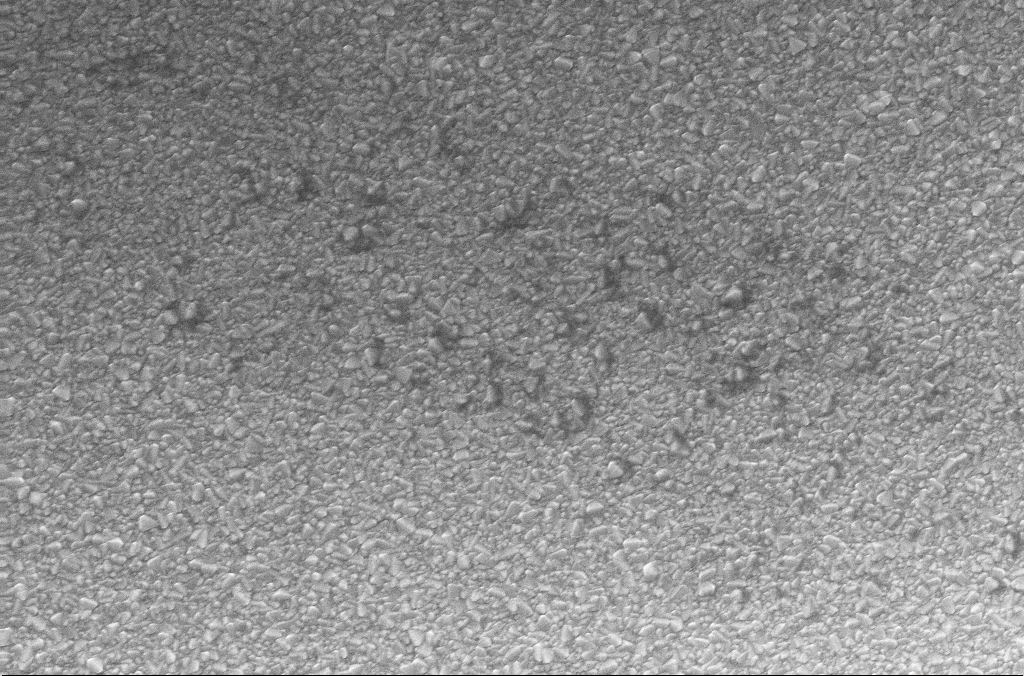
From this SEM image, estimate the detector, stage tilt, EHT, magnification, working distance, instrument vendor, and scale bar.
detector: InLens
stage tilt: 0°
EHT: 20 kV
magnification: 15 K X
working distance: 1.9 mm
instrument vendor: Zeiss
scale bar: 2000 nm